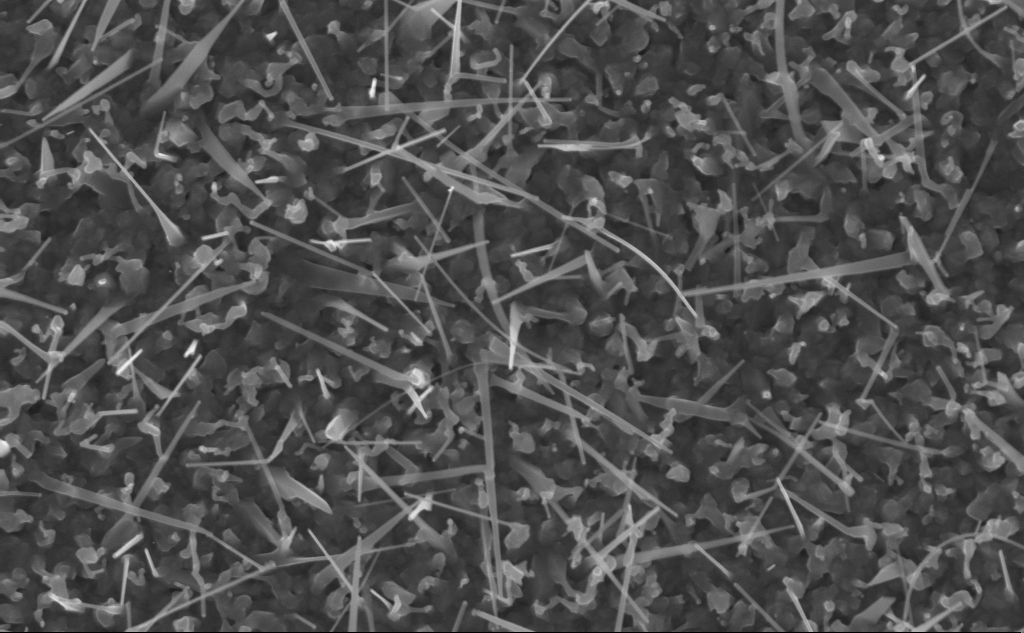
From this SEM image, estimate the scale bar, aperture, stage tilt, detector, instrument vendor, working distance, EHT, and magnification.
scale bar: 1000 nm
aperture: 30 µm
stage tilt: -0°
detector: InLens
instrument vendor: Zeiss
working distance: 5 mm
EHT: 10 kV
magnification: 40 K X